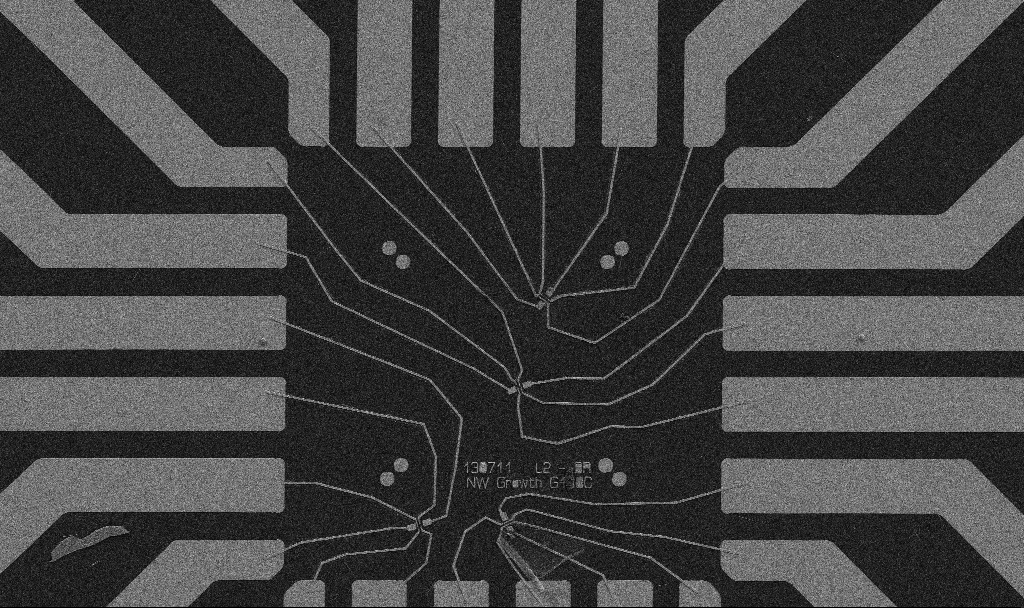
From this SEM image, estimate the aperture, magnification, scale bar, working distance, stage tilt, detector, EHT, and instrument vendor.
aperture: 30 µm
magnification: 1 K X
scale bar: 20000 nm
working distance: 10.7 mm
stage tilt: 0°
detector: SE2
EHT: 5 kV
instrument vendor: Zeiss